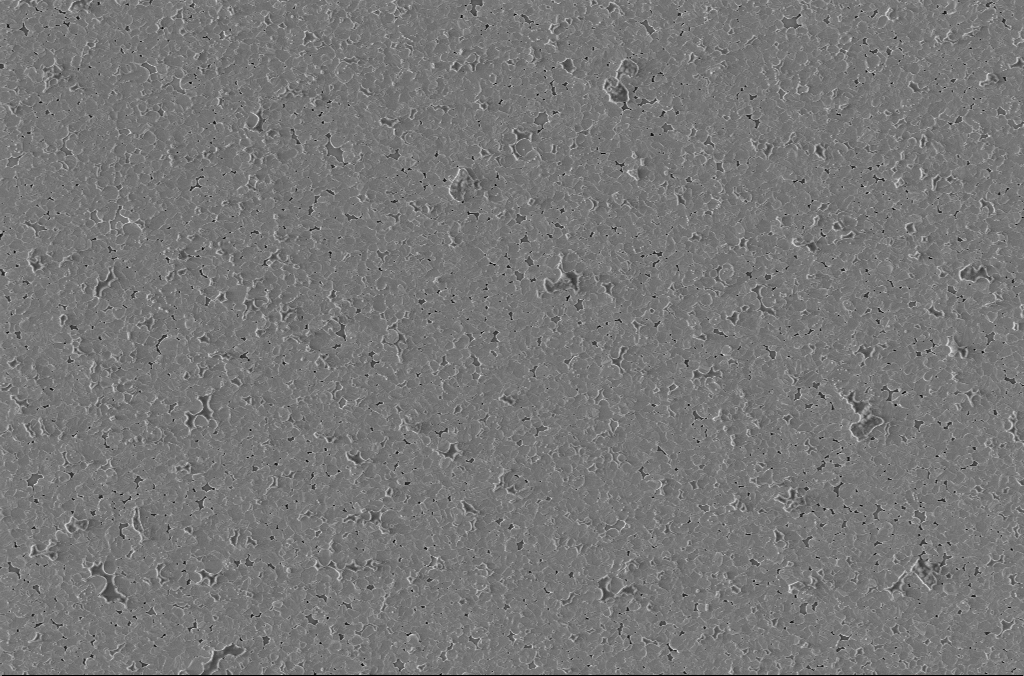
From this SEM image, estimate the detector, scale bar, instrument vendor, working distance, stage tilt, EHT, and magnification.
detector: InLens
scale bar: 10000 nm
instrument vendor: Zeiss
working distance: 3 mm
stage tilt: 0°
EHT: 2 kV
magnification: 5 K X